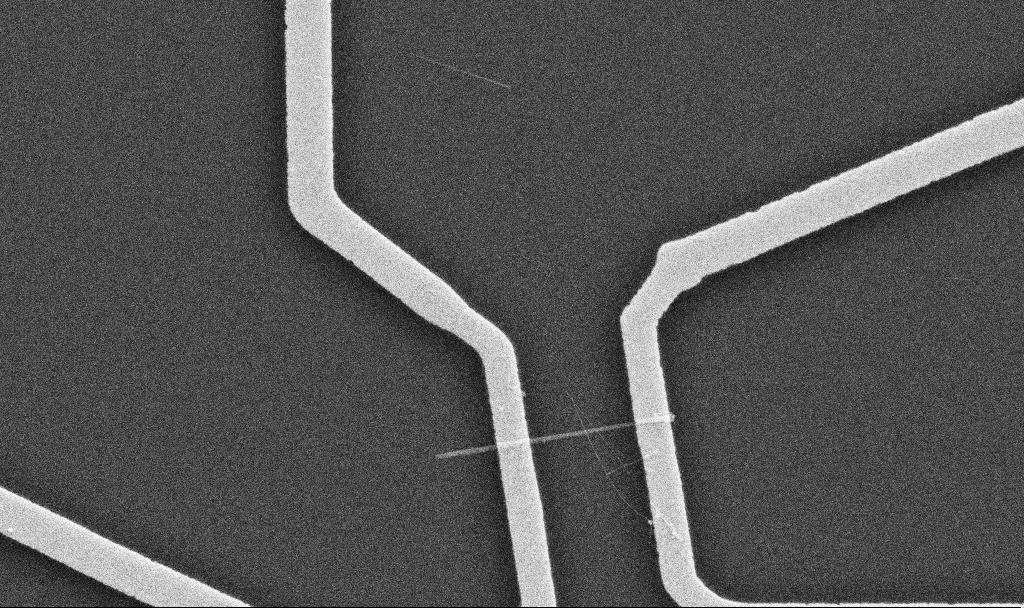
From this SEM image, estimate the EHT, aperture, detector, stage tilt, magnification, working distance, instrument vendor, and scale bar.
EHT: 10 kV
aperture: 30 µm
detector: SE2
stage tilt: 0°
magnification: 20 K X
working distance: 10.7 mm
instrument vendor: Zeiss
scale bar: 1000 nm